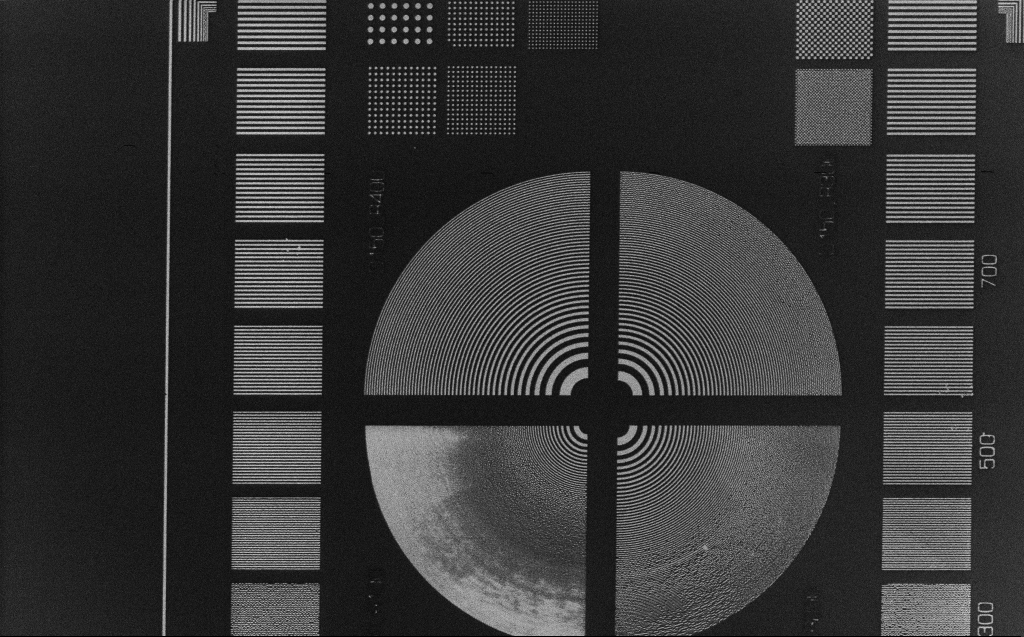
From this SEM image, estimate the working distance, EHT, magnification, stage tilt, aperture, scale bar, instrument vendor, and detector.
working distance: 4 mm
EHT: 3 kV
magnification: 1.1 K X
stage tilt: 0°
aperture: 30 µm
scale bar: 20000 nm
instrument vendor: Zeiss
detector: InLens